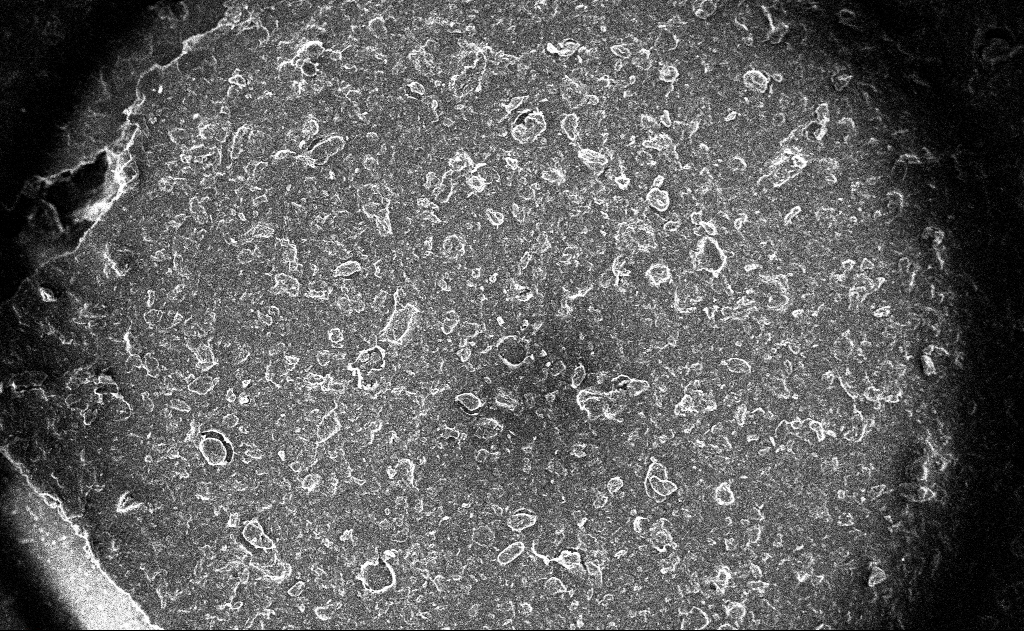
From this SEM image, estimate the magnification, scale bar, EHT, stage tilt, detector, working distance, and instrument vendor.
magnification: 0.134 K X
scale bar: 100000 nm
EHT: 10 kV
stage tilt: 0°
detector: InLens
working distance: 3 mm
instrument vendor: Zeiss